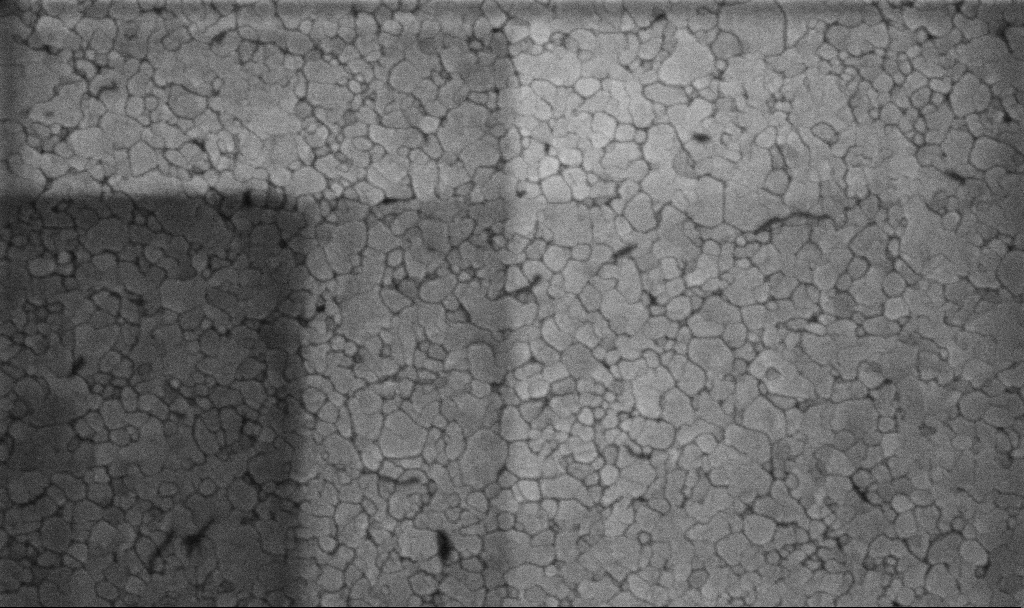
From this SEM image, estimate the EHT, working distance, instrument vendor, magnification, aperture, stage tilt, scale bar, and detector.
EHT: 5 kV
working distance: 3.1 mm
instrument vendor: Zeiss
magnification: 100.15 K X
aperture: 30 µm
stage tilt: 0°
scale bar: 200 nm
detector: InLens